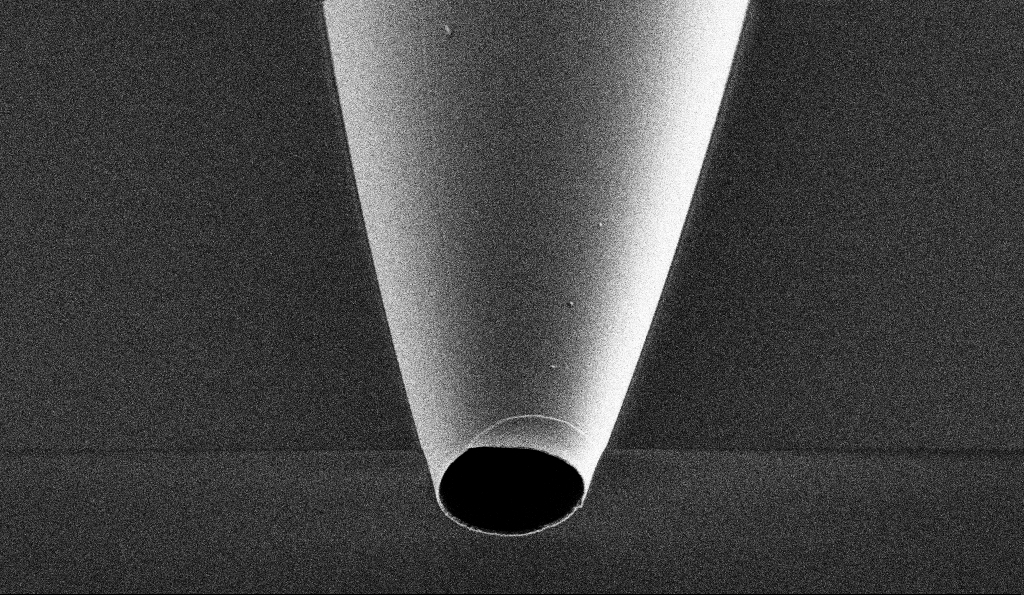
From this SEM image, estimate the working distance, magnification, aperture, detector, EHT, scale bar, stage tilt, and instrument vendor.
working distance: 6.6 mm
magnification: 25 K X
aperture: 30 µm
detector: SE2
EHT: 1 kV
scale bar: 2000 nm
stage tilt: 45°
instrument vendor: Zeiss